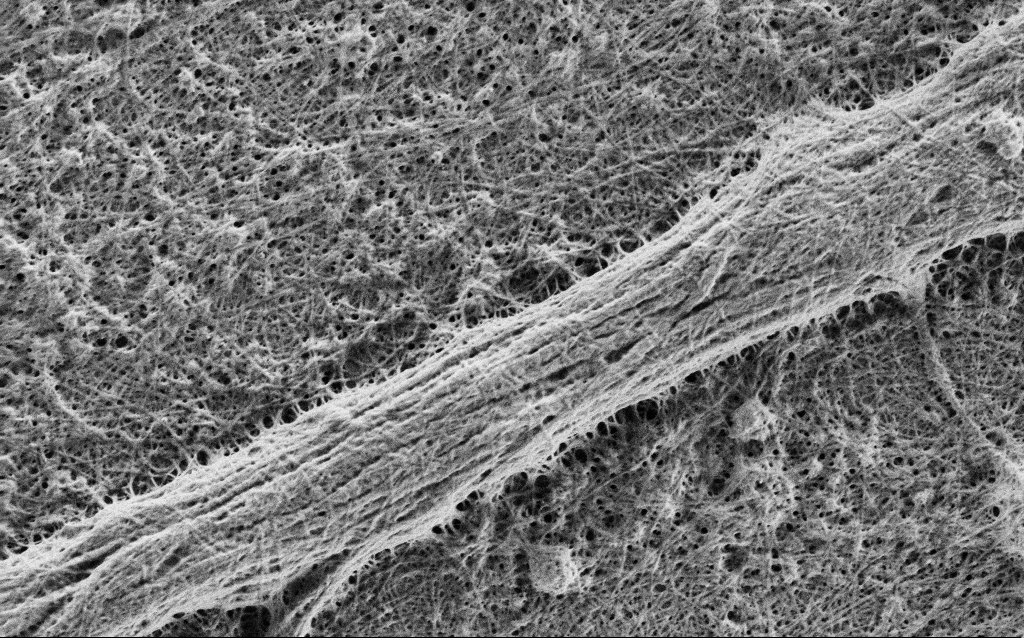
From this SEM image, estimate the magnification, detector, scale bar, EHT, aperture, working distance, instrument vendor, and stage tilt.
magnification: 25 K X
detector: SE2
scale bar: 2000 nm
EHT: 1 kV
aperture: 30 µm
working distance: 4 mm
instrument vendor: Zeiss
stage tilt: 0°